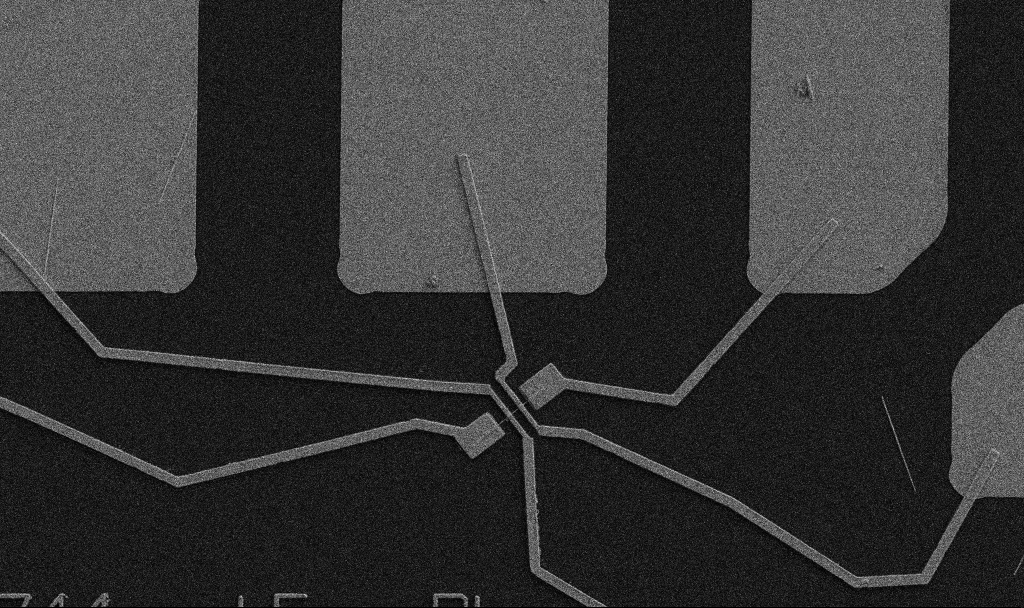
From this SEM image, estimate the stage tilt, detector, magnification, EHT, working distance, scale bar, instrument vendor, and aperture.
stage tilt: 0°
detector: SE2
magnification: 5 K X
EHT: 5 kV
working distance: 10.7 mm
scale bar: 10000 nm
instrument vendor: Zeiss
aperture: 30 µm